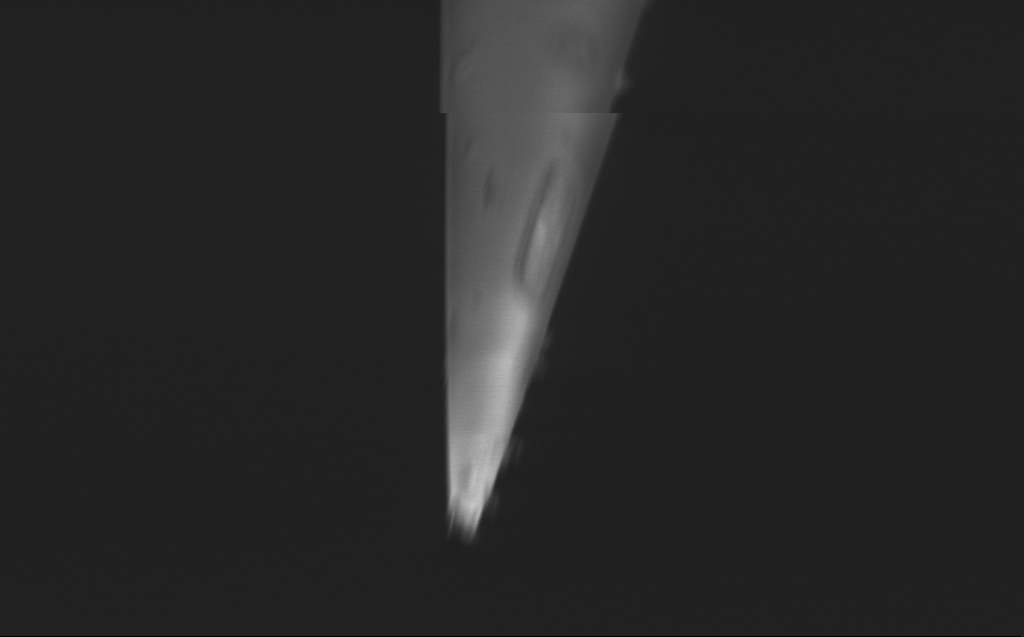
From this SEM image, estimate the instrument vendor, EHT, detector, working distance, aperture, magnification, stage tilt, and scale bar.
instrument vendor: Zeiss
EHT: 1 kV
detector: InLens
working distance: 3 mm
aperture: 30 µm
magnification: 53.49 K X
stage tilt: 45°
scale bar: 1000 nm